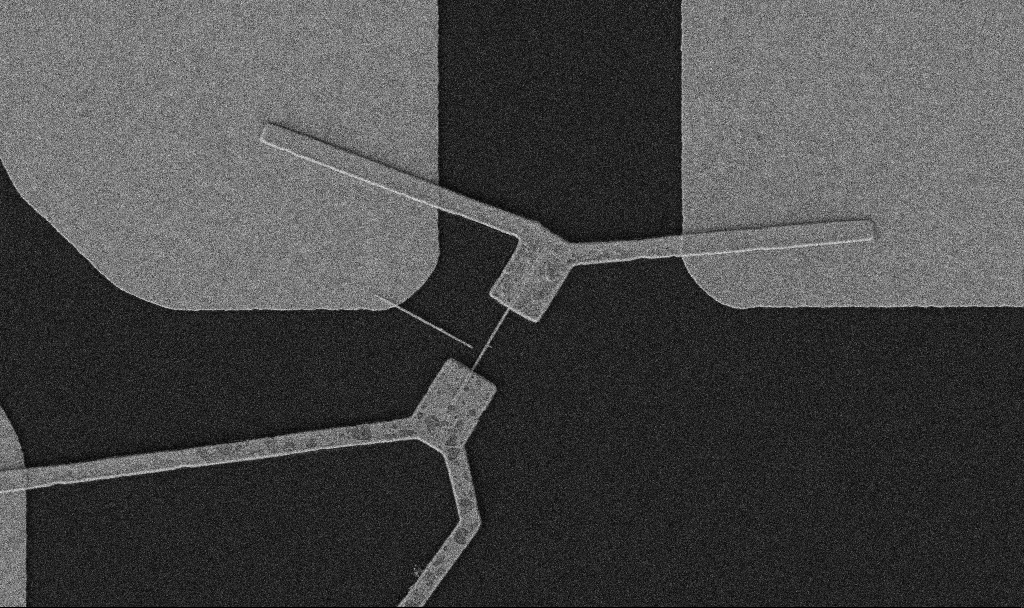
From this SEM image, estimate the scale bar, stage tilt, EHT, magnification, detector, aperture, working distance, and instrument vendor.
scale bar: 2000 nm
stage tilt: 0°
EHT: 5 kV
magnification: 10 K X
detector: SE2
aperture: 30 µm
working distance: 10.7 mm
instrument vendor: Zeiss